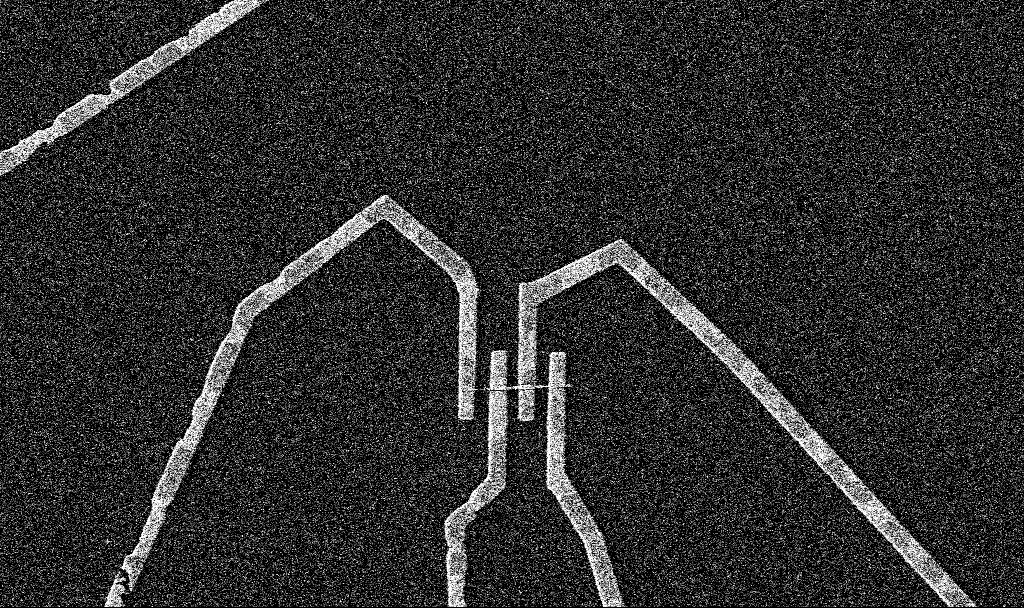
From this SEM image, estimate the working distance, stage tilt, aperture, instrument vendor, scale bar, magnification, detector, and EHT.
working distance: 8.5 mm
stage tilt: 0°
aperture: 30 µm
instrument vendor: Zeiss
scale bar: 2000 nm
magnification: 9.95 K X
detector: SE2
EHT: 5 kV